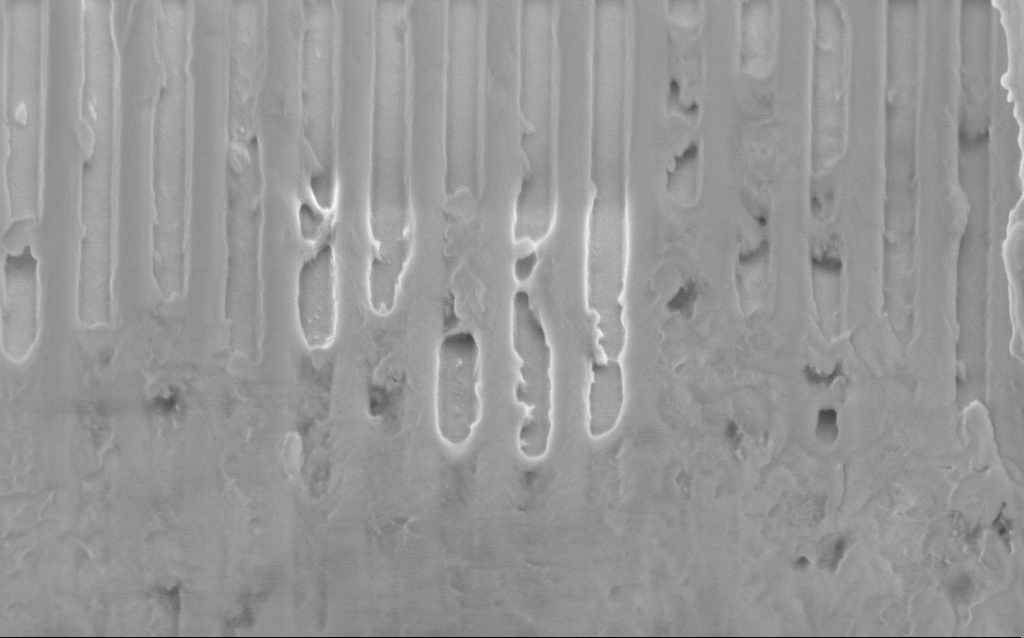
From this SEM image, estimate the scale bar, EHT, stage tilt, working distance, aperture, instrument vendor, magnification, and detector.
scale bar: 1000 nm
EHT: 2 kV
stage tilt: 45°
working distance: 2.7 mm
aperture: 30 µm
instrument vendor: Zeiss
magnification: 54.09 K X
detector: InLens